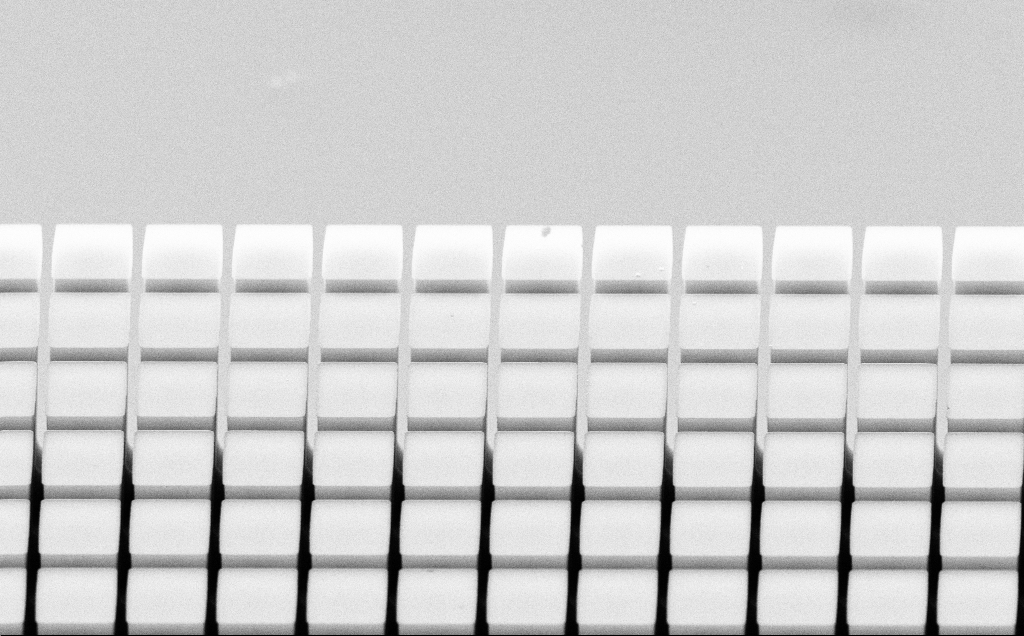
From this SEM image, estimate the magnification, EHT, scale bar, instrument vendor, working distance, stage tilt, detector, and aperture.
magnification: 6.6 K X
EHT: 20 kV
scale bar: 10000 nm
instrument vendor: Zeiss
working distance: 7 mm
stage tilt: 60°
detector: SE2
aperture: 30 µm